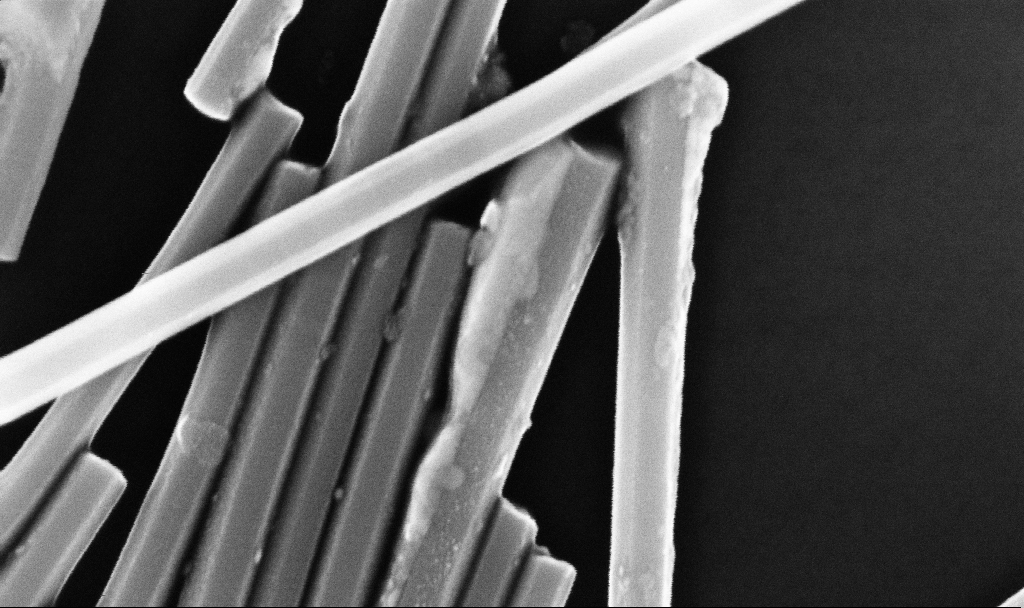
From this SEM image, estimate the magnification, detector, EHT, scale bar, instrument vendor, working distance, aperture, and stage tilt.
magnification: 202.09 K X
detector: InLens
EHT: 10 kV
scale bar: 200 nm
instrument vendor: Zeiss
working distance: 6.7 mm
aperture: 30 µm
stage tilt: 0°